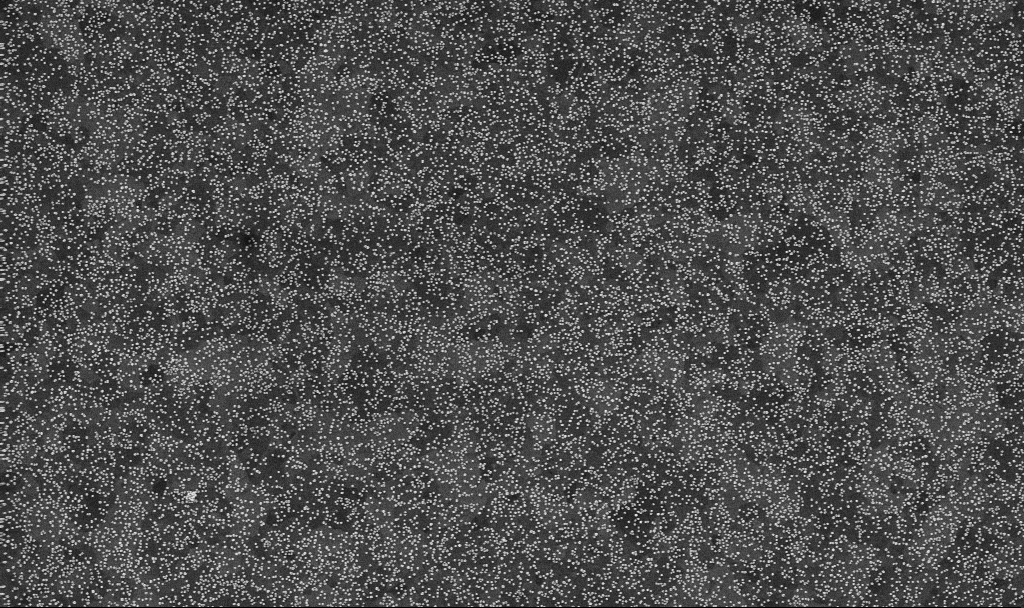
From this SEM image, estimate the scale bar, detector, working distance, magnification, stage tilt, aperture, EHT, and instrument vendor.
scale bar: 1000 nm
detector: InLens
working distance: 3.3 mm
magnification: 50 K X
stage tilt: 0°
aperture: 30 µm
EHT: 10 kV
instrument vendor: Zeiss